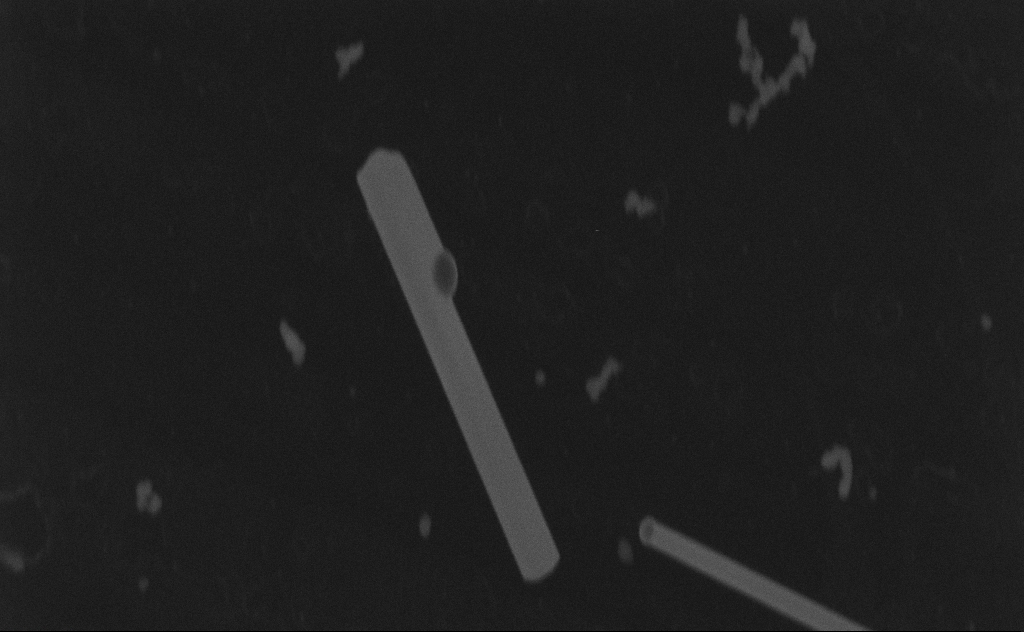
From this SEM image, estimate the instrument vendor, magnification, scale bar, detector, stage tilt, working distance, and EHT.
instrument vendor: Zeiss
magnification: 134.99 K X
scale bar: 200 nm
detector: SE2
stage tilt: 0°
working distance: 8 mm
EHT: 20 kV